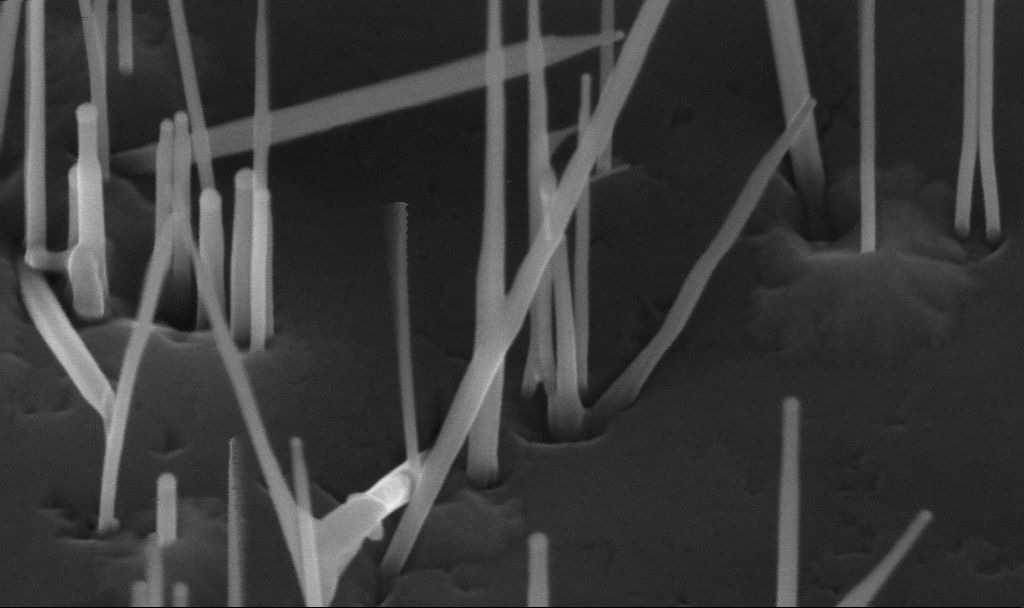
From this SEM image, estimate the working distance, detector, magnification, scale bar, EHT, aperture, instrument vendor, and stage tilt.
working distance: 5.6 mm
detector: InLens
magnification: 200 K X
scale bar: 200 nm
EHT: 10 kV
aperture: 30 µm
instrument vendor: Zeiss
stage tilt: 45°